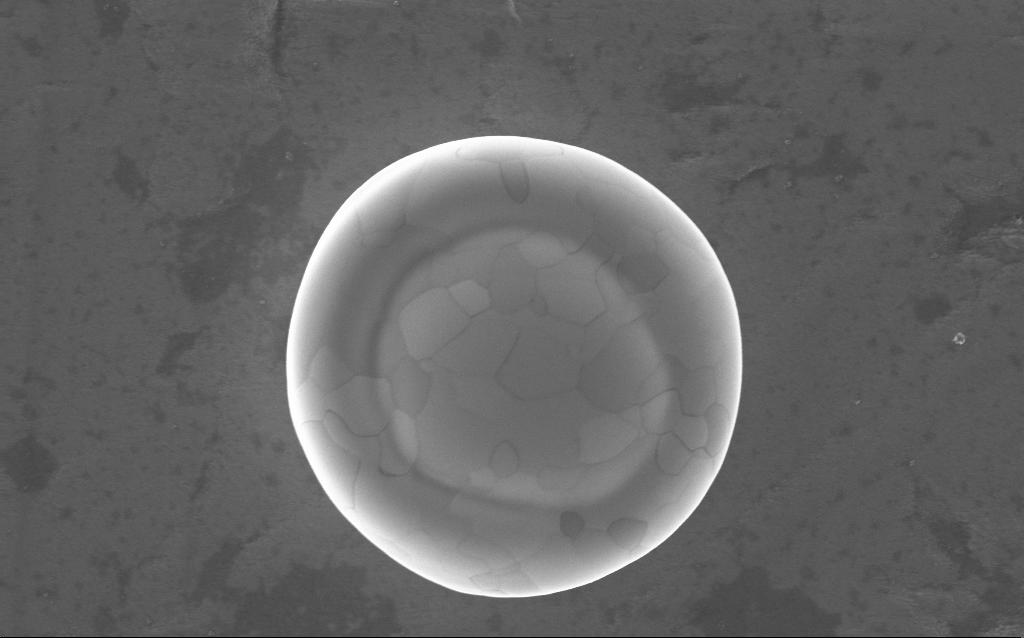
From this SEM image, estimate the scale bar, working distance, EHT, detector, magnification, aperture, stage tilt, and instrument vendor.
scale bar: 1000 nm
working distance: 2 mm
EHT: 5 kV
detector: InLens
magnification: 40 K X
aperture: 30 µm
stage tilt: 0°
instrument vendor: Zeiss